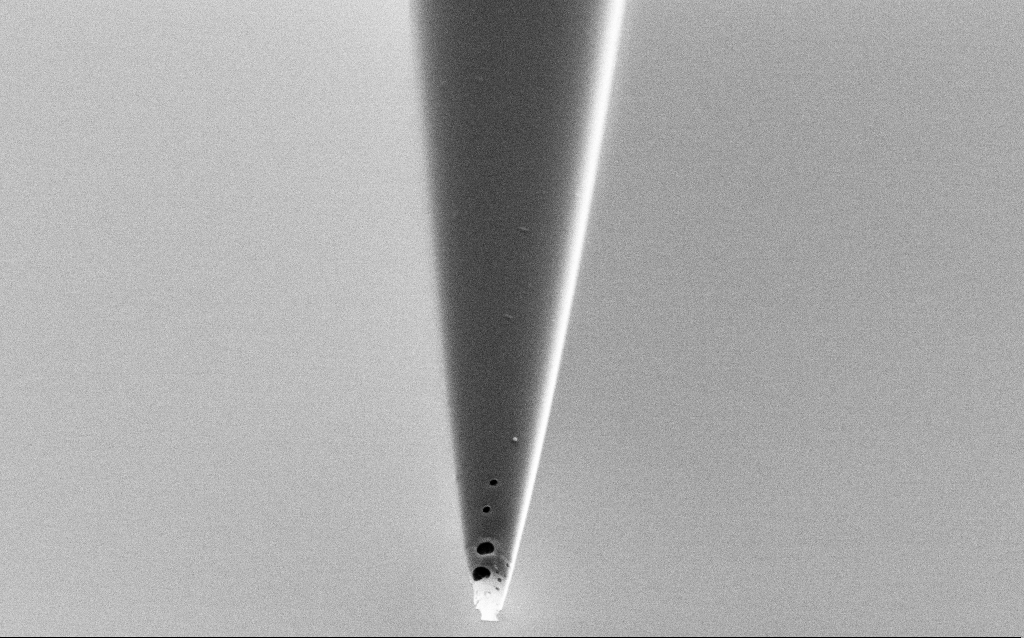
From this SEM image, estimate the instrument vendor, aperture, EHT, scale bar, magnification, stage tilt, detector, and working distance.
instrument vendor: Zeiss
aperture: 30 µm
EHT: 2 kV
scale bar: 2000 nm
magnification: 28.28 K X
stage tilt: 45°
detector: SE2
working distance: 6 mm